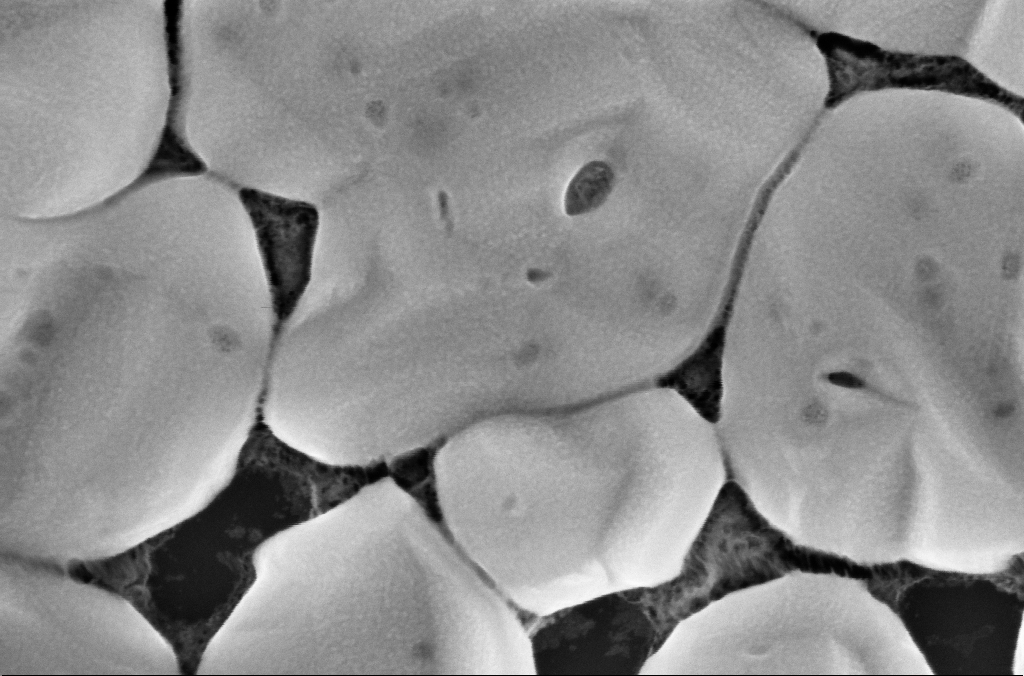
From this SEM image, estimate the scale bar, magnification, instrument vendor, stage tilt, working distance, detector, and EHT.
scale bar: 200 nm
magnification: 200 K X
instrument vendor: Zeiss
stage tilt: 0°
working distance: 3 mm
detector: InLens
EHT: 5 kV